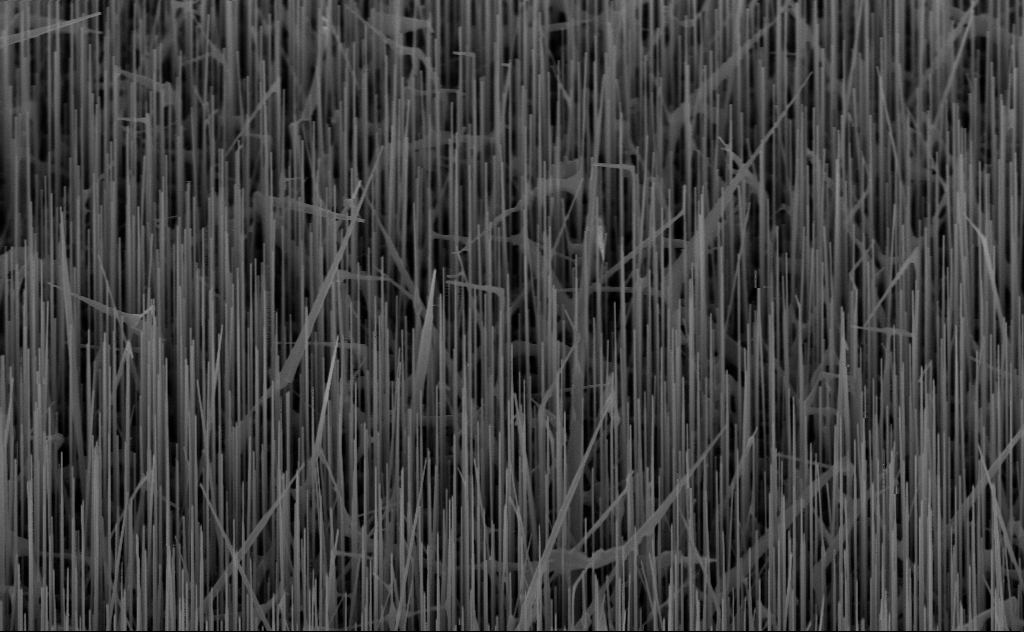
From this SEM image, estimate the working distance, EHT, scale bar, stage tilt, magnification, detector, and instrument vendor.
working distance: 8 mm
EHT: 10 kV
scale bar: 1000 nm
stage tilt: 45°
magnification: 20 K X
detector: InLens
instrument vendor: Zeiss